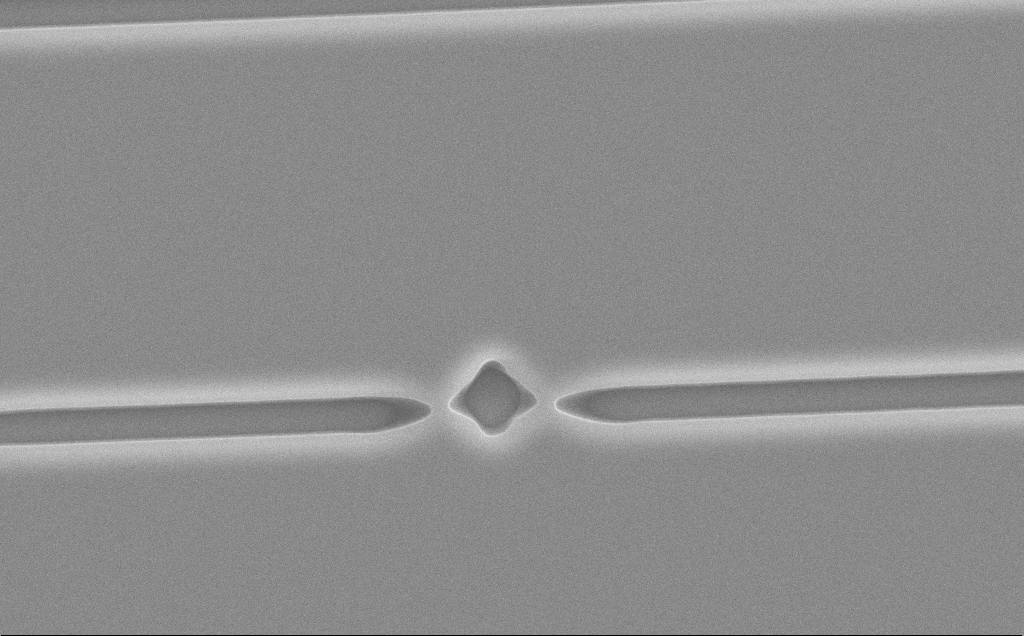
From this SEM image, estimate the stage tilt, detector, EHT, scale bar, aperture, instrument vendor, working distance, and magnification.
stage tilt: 0°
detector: SE2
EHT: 10 kV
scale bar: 2000 nm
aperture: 30 µm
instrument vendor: Zeiss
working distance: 13 mm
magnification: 7.61 K X